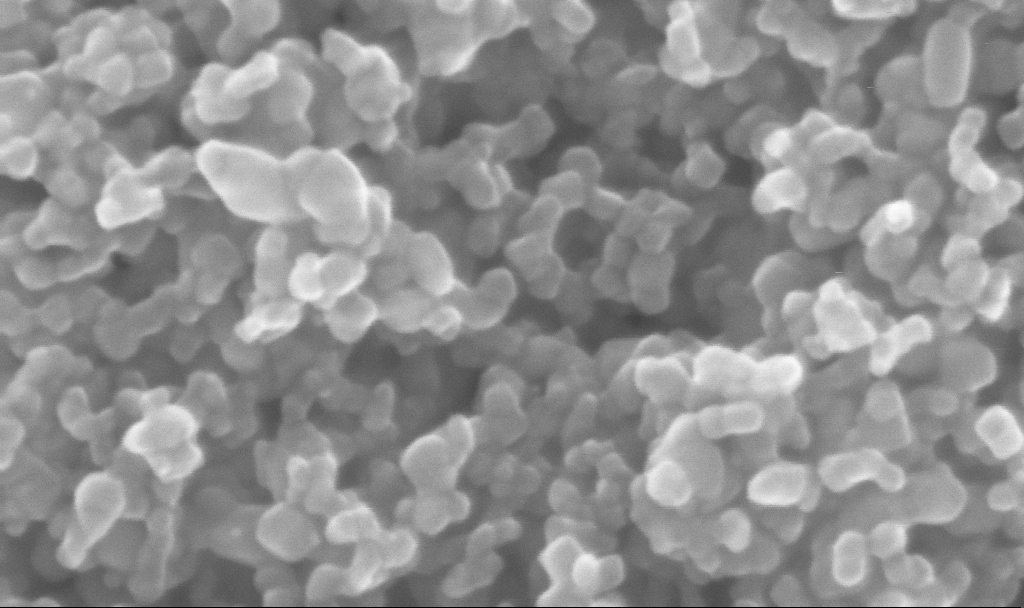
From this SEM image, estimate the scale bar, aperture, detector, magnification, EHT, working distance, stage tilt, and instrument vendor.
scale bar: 100 nm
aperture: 30 µm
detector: InLens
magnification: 479.71 K X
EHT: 10 kV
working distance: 2.7 mm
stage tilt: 0°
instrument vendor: Zeiss